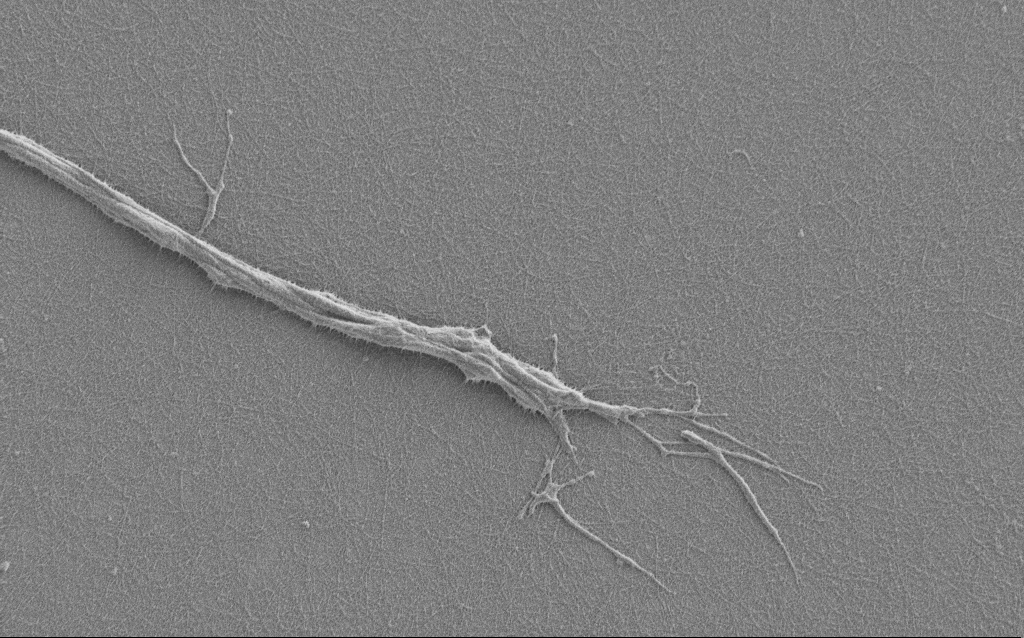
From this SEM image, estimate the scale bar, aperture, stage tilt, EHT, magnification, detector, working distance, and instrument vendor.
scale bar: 2000 nm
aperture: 30 µm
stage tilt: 0°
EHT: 1 kV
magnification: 7.5 K X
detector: SE2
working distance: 6 mm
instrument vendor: Zeiss